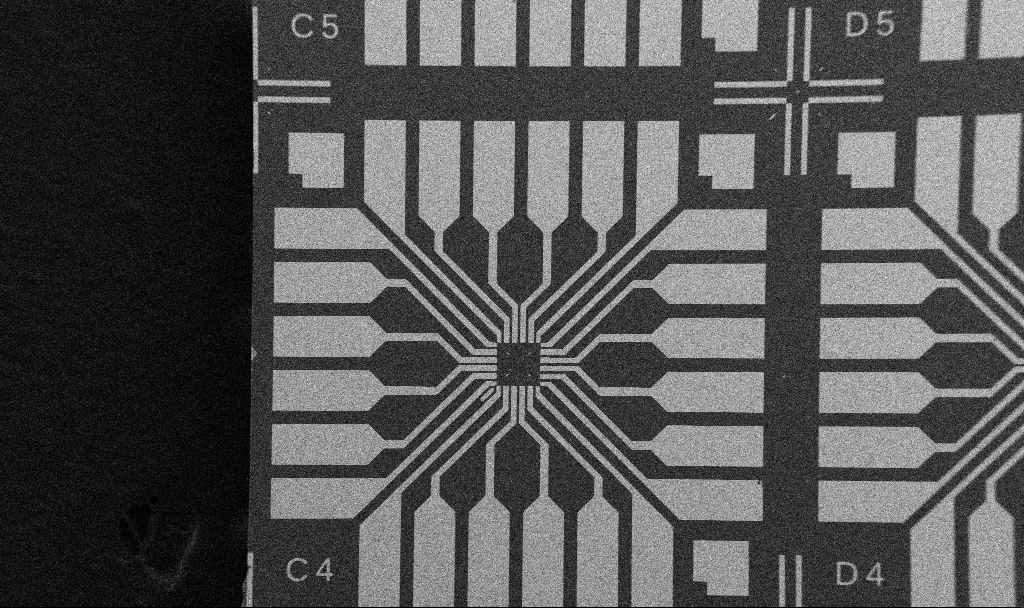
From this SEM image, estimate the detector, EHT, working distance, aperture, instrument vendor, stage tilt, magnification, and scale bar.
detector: SE2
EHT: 5 kV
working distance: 10.7 mm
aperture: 30 µm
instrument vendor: Zeiss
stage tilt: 0°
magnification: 0.1 K X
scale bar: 200000 nm